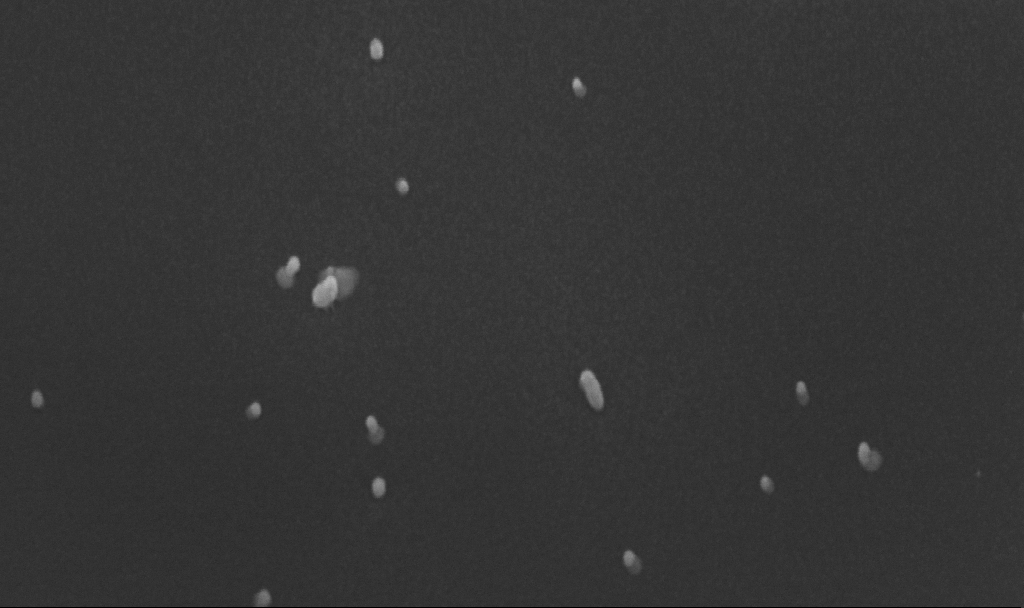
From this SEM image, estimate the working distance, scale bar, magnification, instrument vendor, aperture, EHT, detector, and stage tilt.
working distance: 4.8 mm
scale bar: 100 nm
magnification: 200 K X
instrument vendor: Zeiss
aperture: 30 µm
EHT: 10 kV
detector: InLens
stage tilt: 45°